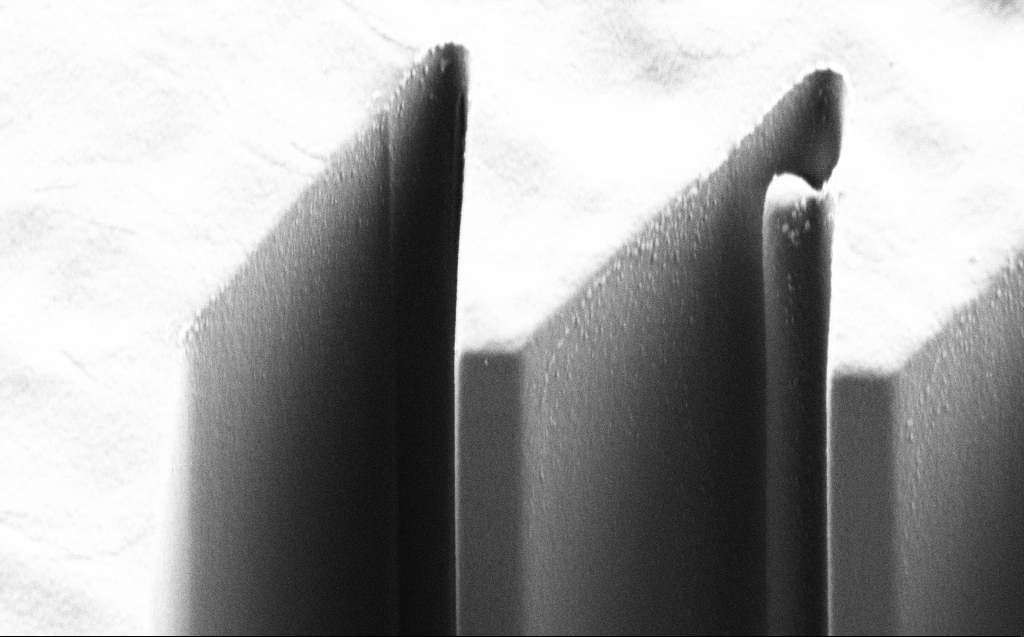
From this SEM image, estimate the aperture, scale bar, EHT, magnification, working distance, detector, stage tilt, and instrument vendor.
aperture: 30 µm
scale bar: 2000 nm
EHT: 1 kV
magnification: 12.02 K X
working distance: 7 mm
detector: SE2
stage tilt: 44.9°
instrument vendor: Zeiss